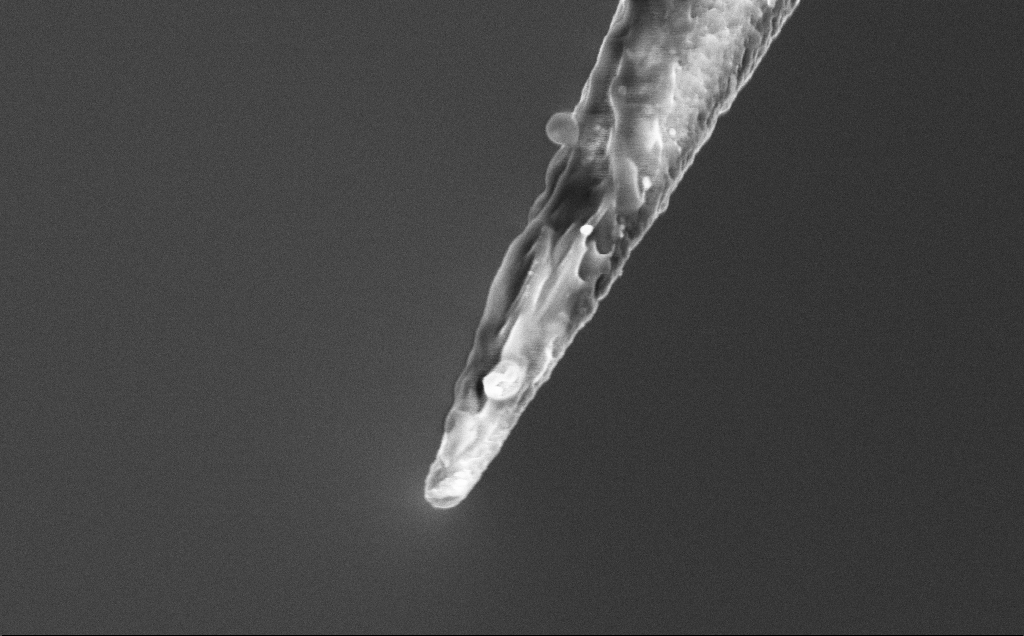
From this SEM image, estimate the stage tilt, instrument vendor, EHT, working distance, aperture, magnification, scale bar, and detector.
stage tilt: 45°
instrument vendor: Zeiss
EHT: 3 kV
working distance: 7.7 mm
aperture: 30 µm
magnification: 50 K X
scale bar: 1000 nm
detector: InLens